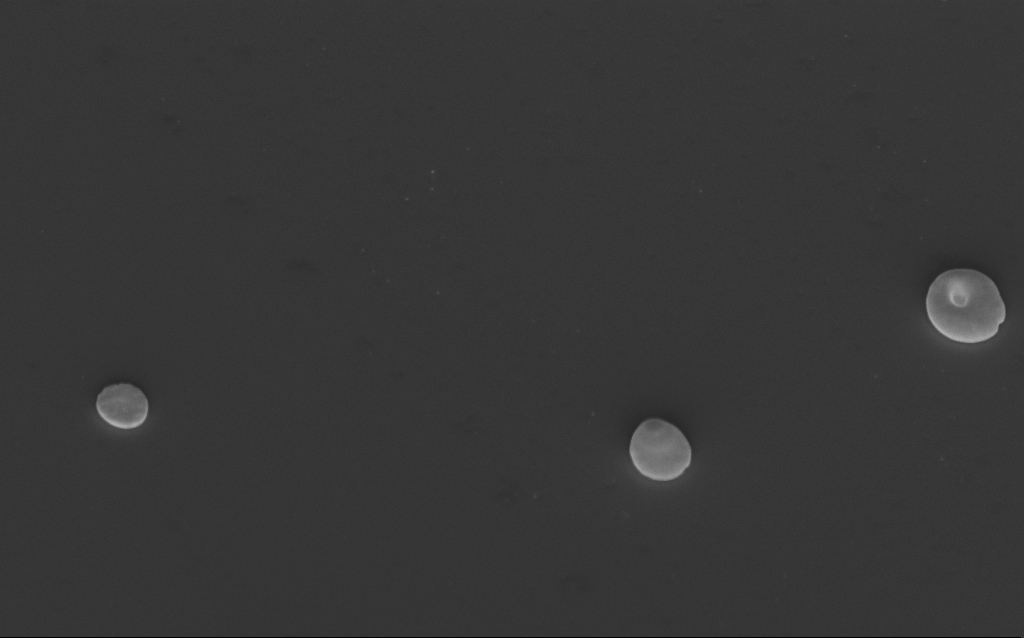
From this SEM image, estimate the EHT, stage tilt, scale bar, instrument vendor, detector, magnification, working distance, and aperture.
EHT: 3 kV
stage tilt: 40°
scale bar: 2000 nm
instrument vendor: Zeiss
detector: InLens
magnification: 25.31 K X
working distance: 5 mm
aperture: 30 µm